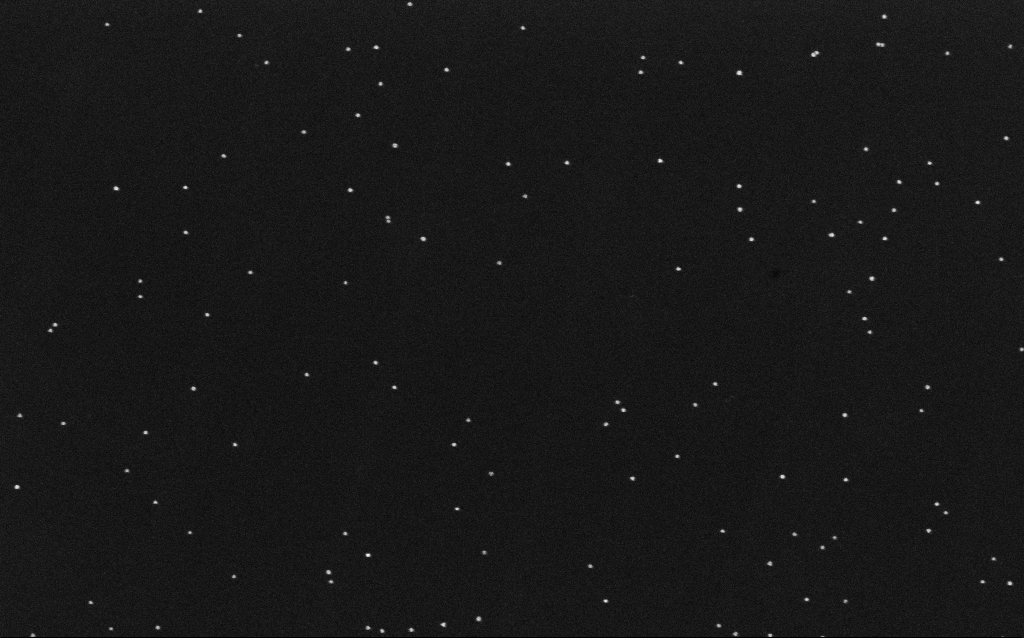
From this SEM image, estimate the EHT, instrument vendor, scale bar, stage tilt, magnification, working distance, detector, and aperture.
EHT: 10 kV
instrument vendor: Zeiss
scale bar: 200 nm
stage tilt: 0°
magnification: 100 K X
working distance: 6.5 mm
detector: InLens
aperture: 30 µm